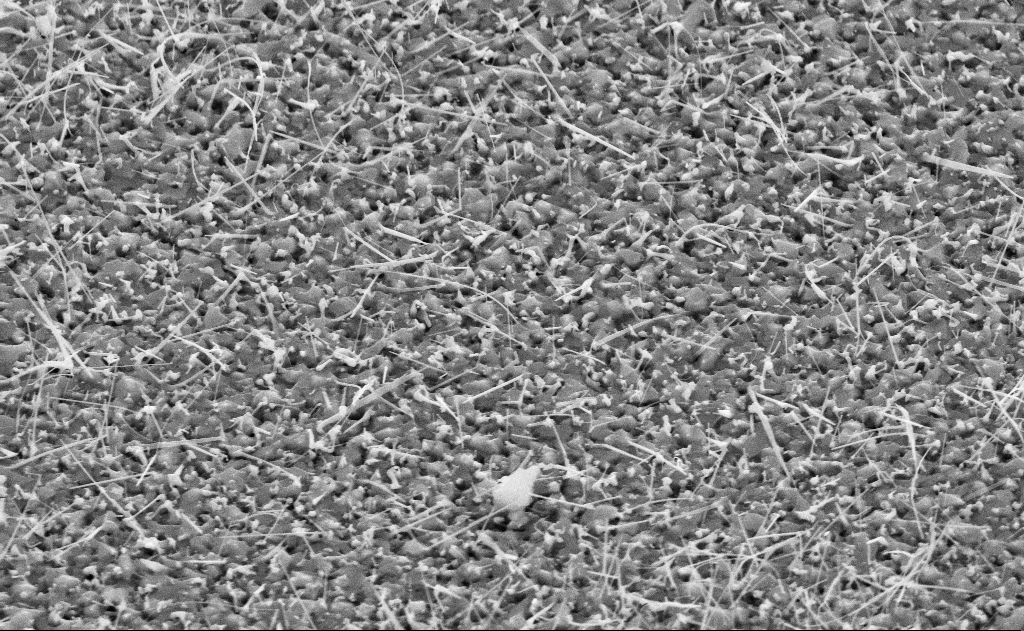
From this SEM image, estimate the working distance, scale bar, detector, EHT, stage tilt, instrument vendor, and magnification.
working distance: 11 mm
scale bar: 2000 nm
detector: SE2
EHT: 10 kV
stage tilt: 45°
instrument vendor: Zeiss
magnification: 20 K X